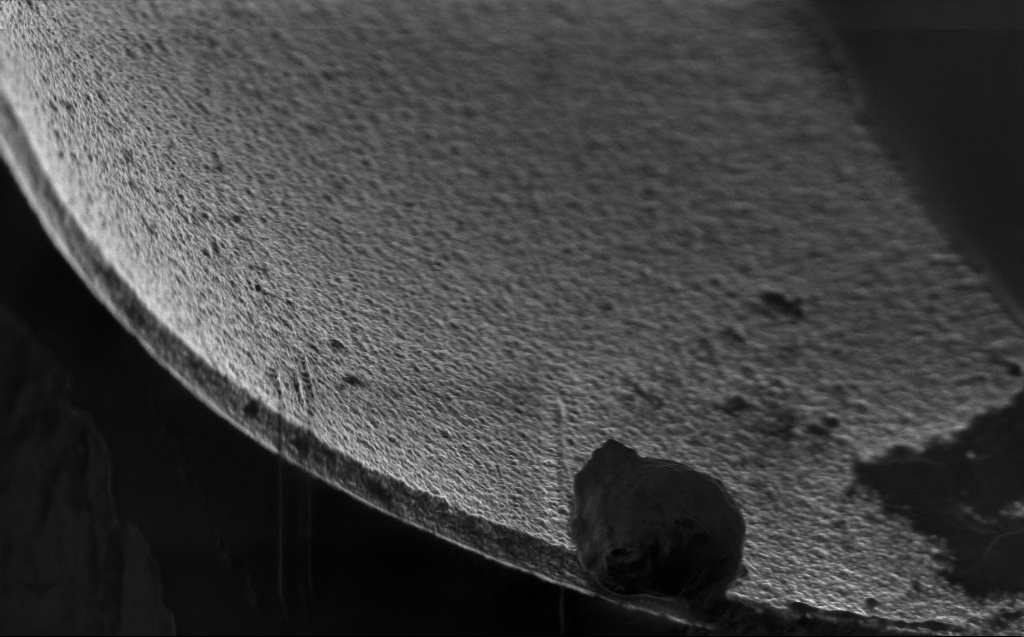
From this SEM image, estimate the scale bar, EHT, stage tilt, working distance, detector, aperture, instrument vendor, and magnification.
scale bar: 2000 nm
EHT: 1 kV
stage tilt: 45°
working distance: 3 mm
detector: InLens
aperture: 30 µm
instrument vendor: Zeiss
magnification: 23.53 K X